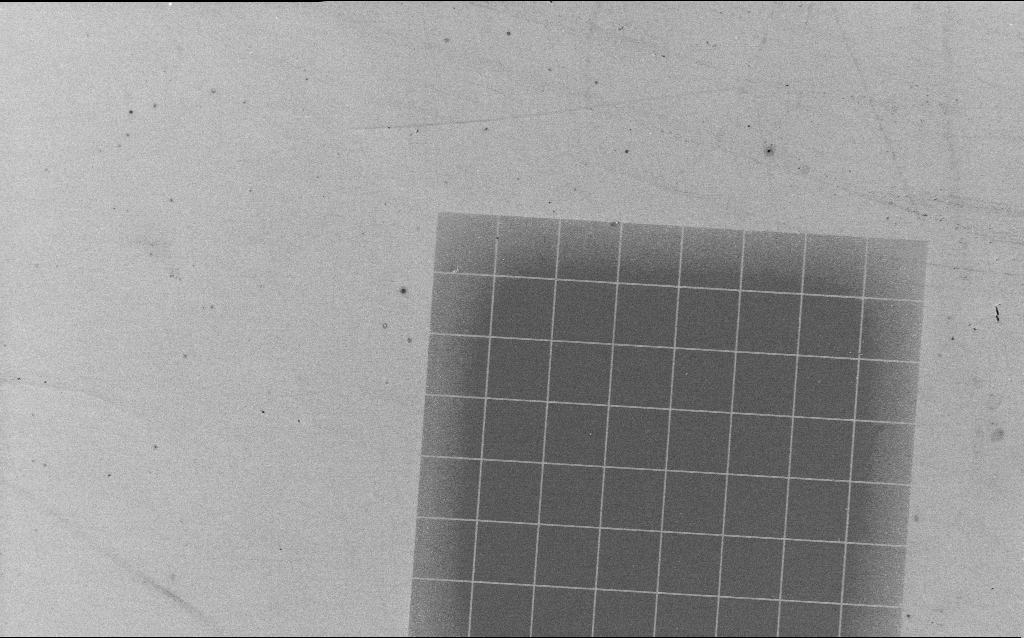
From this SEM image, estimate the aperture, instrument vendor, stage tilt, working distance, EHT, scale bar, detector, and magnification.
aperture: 30 µm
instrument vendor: Zeiss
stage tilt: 0°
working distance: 4.4 mm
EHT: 3 kV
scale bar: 20000 nm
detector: InLens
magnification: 1.01 K X